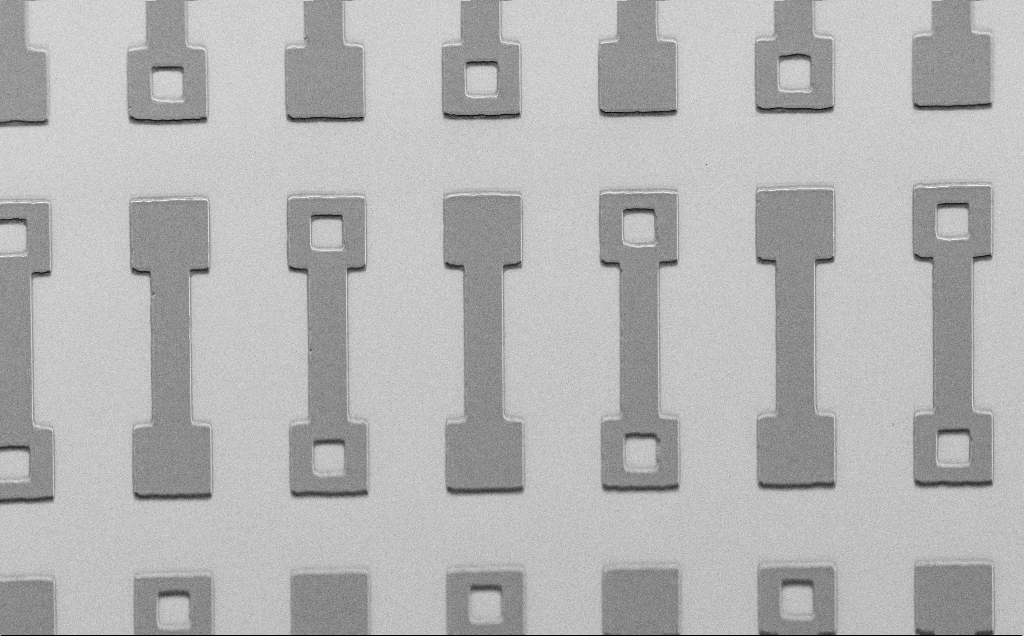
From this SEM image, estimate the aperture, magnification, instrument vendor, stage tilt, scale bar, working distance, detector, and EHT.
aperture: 30 µm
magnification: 0.572 K X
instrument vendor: Zeiss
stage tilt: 45°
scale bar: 100000 nm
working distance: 7 mm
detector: SE2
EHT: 1.5 kV